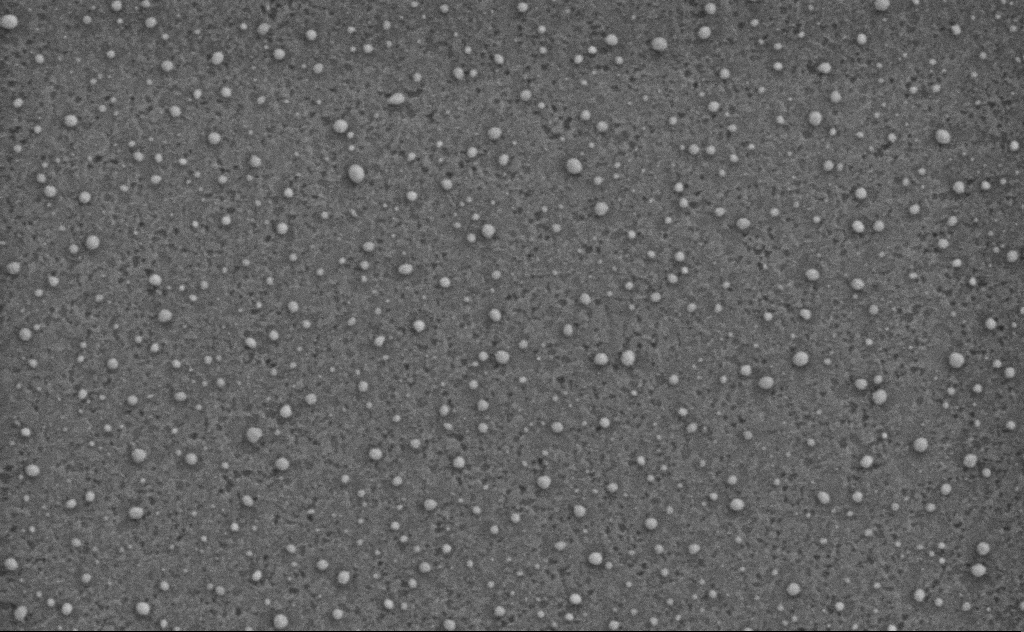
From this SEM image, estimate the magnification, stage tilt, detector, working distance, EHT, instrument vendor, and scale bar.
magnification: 80 K X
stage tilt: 0°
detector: SE2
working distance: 4 mm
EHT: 3 kV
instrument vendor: Zeiss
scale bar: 200 nm